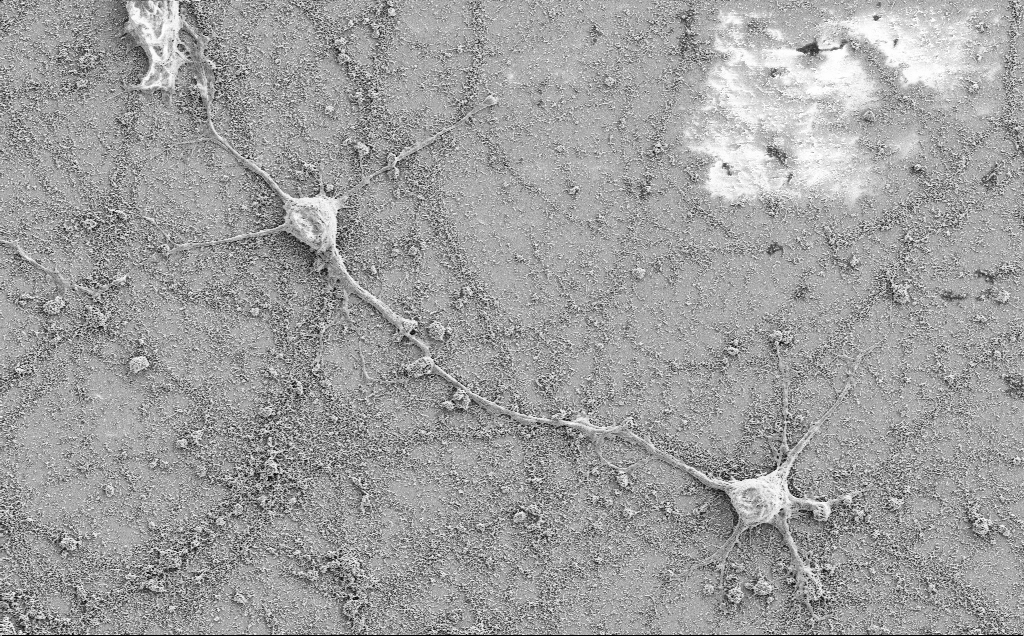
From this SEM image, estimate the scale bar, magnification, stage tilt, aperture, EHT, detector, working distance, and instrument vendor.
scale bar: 10000 nm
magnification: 3 K X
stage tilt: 0°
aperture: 30 µm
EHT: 2 kV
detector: SE2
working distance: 7.1 mm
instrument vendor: Zeiss